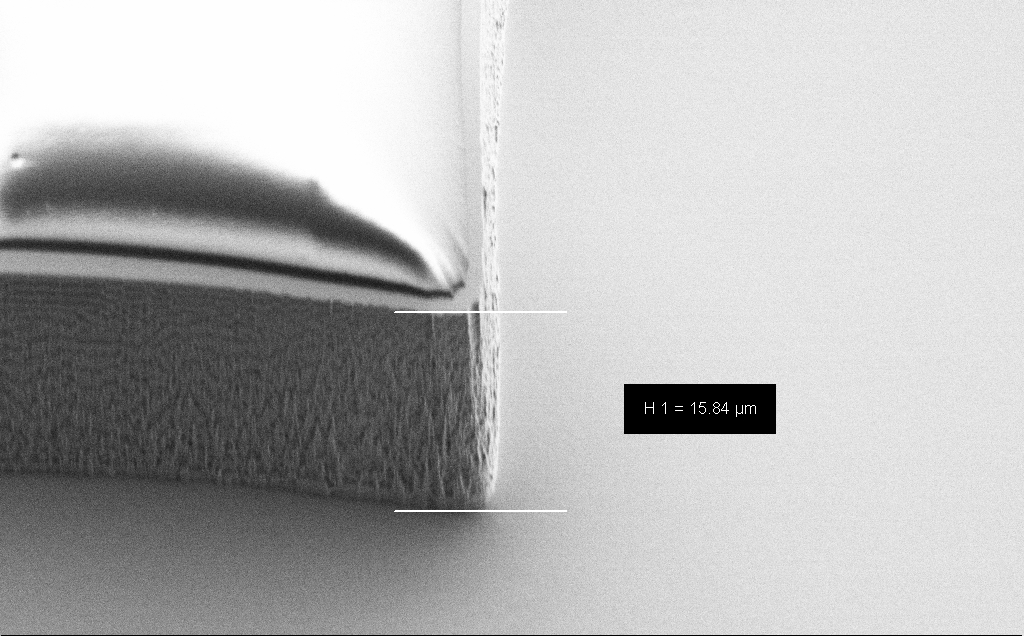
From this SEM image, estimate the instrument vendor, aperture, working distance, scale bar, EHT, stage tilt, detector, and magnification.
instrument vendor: Zeiss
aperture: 30 µm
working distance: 7 mm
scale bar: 10000 nm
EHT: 1.2 kV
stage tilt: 45°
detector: SE2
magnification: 4.61 K X